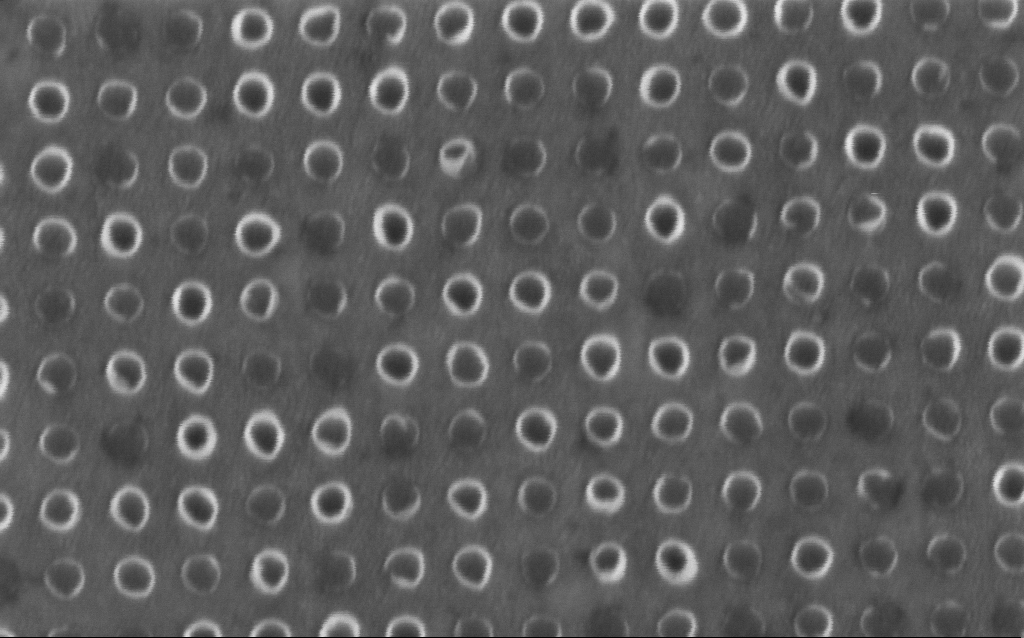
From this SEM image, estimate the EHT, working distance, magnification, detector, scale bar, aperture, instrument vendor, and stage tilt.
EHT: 1.5 kV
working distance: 5.9 mm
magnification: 176.55 K X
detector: InLens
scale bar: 200 nm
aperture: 30 µm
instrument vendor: Zeiss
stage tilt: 0°